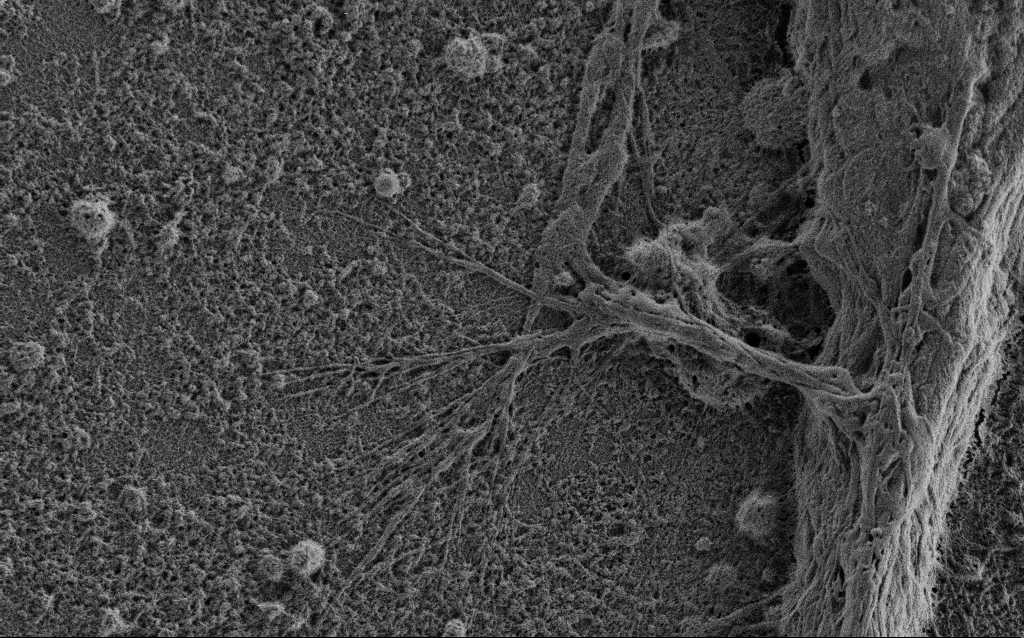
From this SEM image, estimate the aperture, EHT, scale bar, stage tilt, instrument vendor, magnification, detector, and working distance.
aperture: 30 µm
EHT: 0.9 kV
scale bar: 2000 nm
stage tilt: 0°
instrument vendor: Zeiss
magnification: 10 K X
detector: SE2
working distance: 3.4 mm